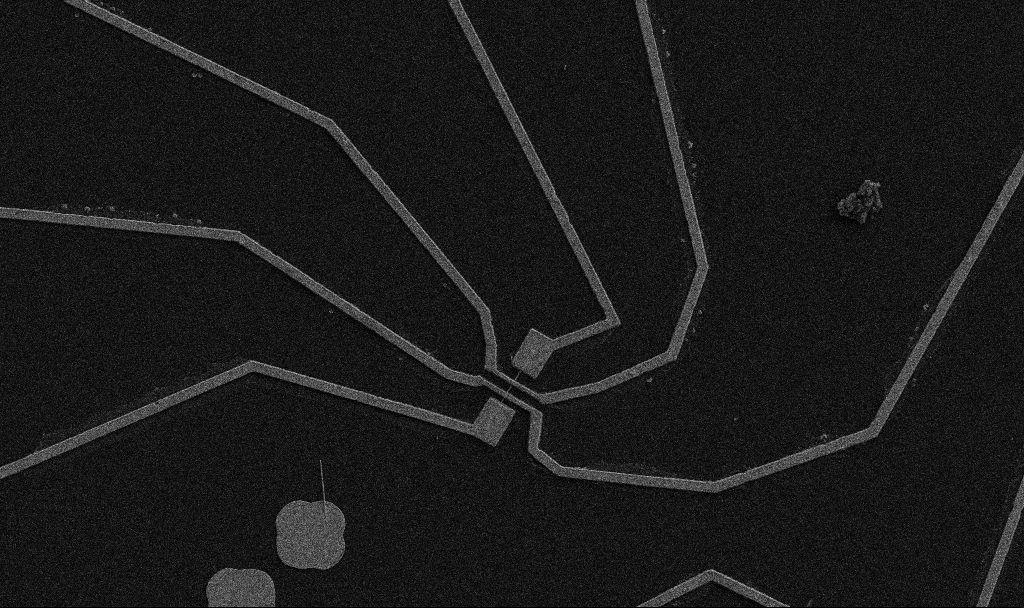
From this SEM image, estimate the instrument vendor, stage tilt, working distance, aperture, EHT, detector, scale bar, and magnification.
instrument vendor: Zeiss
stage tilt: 0°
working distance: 10.7 mm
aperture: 30 µm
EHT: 5 kV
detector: SE2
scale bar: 10000 nm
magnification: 5 K X